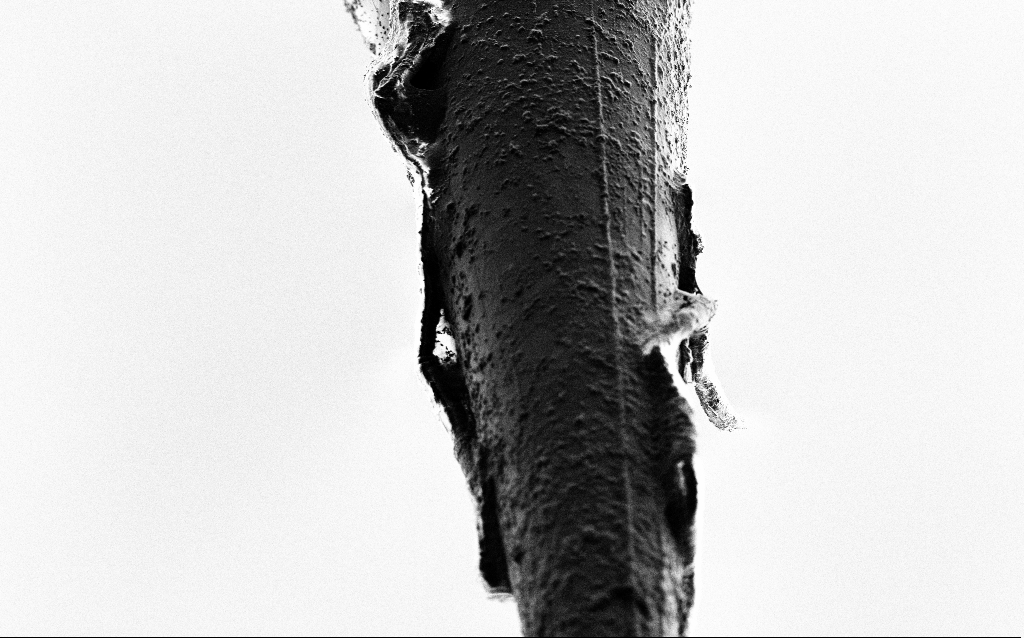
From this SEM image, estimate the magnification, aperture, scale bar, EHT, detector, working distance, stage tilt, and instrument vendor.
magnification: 0.5 K X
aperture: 30 µm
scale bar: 100000 nm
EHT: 5 kV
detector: SE2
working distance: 5.8 mm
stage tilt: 43.9°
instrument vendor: Zeiss